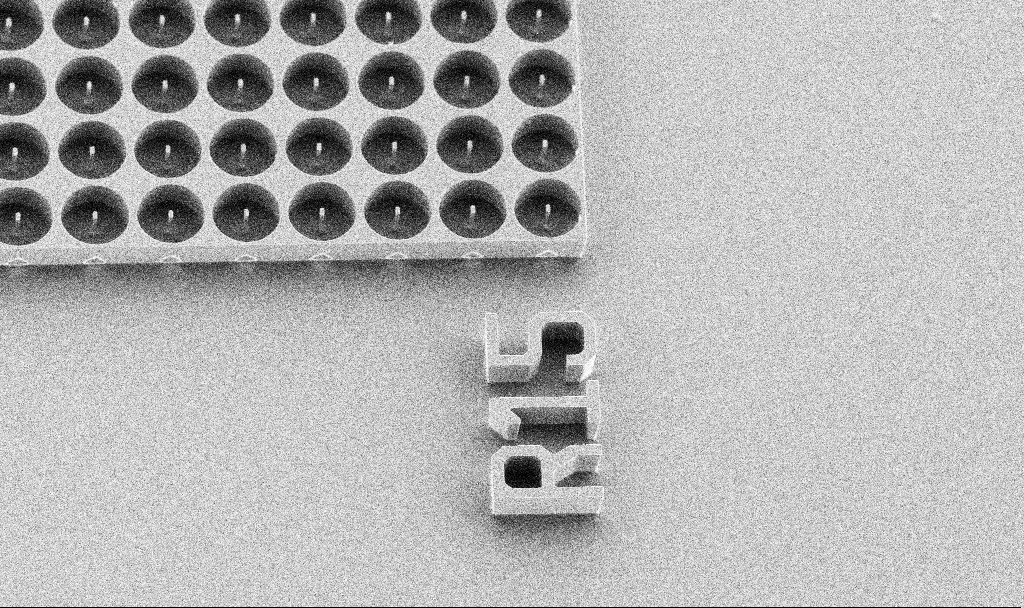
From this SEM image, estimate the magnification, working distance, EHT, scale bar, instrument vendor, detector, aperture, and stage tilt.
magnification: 0.846 K X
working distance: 7.4 mm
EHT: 5 kV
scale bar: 20000 nm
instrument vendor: Zeiss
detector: SE2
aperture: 30 µm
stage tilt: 30°